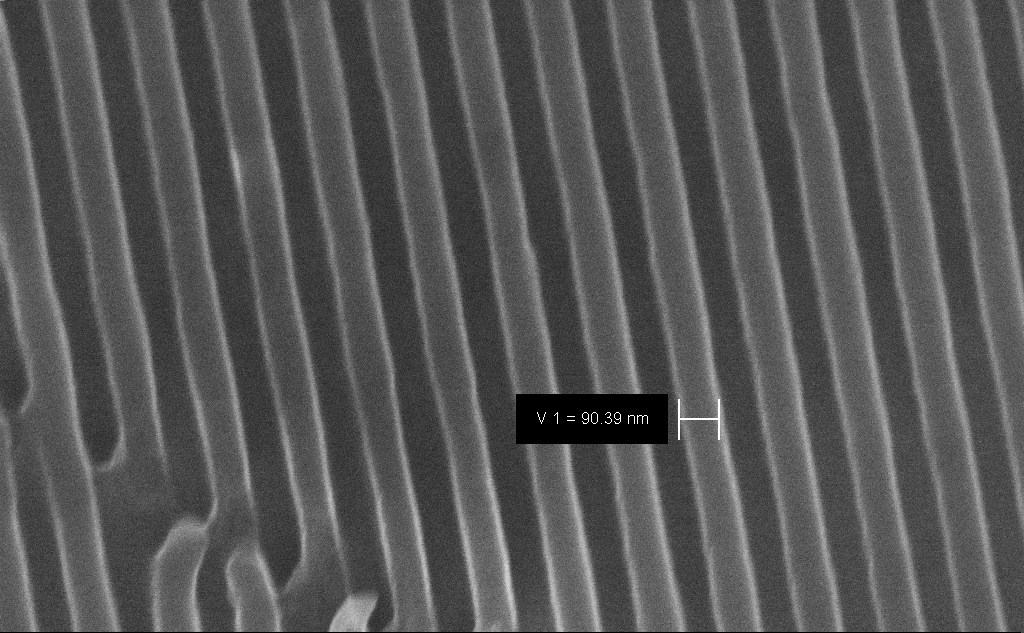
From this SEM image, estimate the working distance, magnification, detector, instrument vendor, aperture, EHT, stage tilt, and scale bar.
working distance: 7.9 mm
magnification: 162.49 K X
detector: InLens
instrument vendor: Zeiss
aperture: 30 µm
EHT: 10 kV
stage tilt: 45°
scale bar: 200 nm